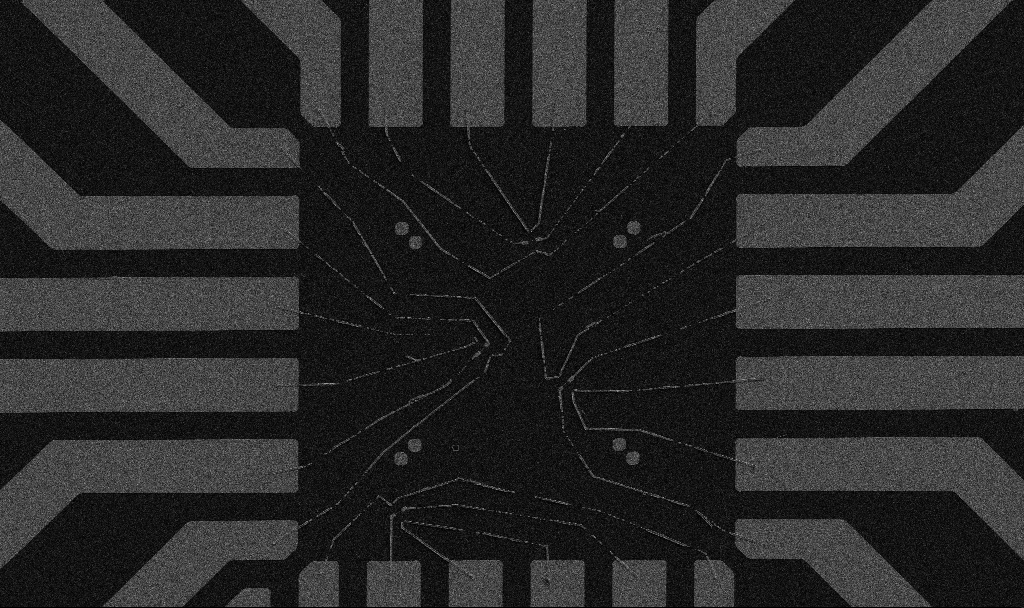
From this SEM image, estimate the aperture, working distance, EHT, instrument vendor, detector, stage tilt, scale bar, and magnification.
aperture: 30 µm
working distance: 10.7 mm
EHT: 5 kV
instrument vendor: Zeiss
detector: SE2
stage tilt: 0°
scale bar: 20000 nm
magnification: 1 K X